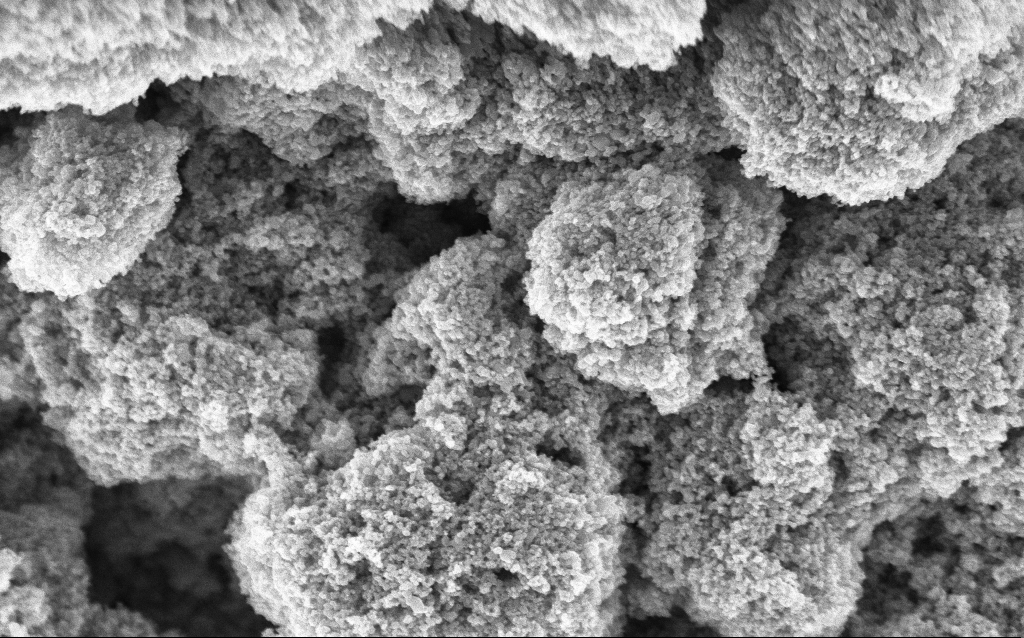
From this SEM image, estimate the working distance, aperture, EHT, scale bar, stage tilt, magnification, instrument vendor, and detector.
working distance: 4.6 mm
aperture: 30 µm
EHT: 5 kV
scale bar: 1000 nm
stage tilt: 0°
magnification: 68.7 K X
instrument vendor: Zeiss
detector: InLens